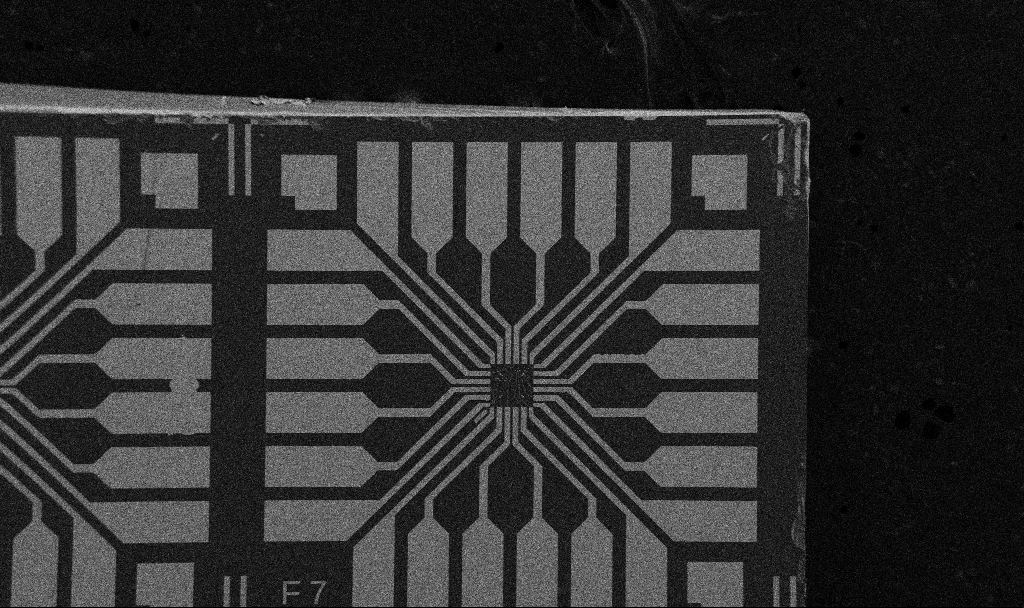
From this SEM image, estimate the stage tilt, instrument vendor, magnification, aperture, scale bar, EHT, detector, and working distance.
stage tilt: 0°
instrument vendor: Zeiss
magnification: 0.1 K X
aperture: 30 µm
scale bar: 200000 nm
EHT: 5 kV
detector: SE2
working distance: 10.7 mm